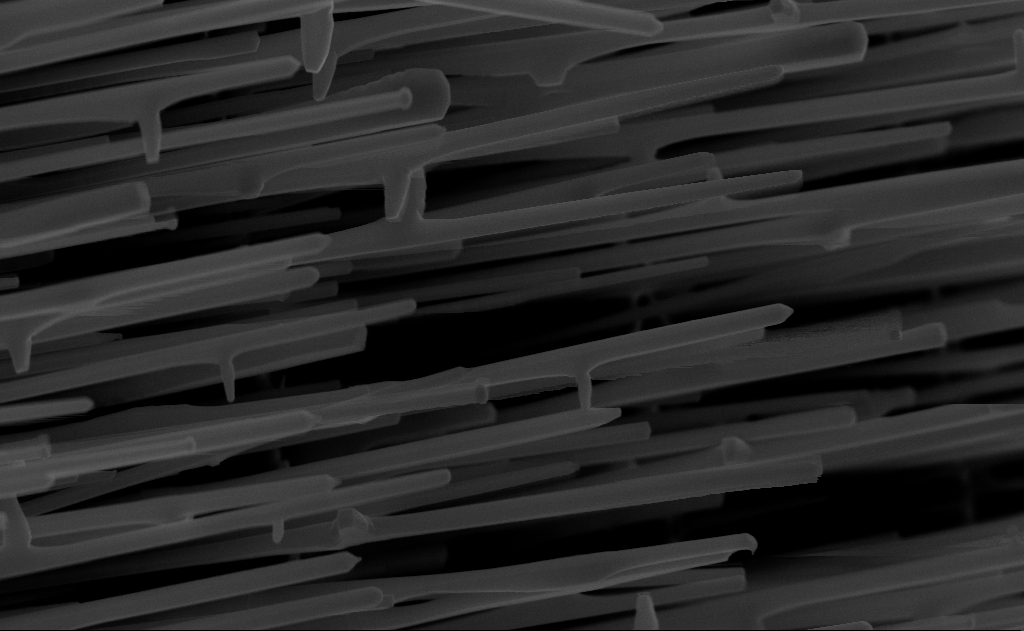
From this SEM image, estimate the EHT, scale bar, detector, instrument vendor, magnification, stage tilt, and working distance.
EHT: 10 kV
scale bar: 1000 nm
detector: InLens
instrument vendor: Zeiss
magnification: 40 K X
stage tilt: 0°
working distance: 7 mm